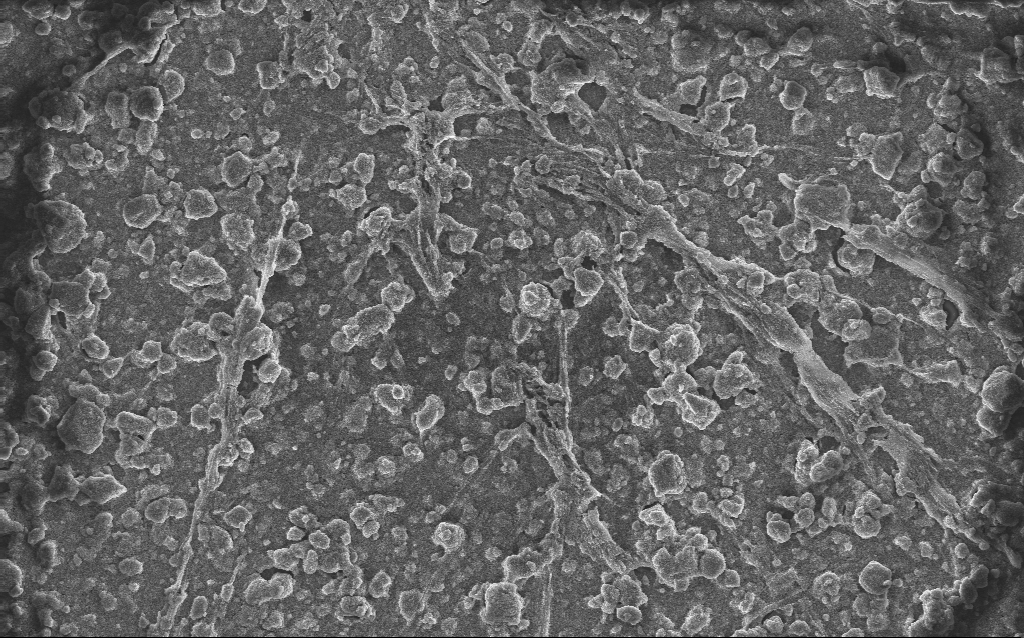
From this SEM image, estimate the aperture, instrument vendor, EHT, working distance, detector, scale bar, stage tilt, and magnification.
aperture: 30 µm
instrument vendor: Zeiss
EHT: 10 kV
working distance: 2.8 mm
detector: InLens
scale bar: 10000 nm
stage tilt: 0°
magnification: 1.69 K X